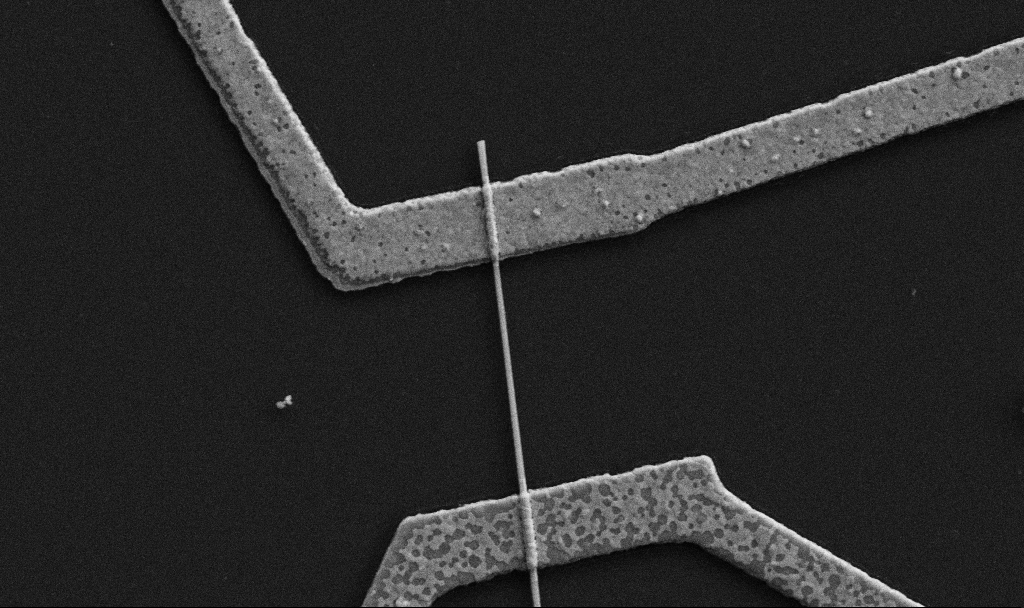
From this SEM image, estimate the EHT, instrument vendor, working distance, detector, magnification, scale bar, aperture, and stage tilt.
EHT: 5 kV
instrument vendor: Zeiss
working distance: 8.7 mm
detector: SE2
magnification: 30 K X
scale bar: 1000 nm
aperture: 30 µm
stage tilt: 0°